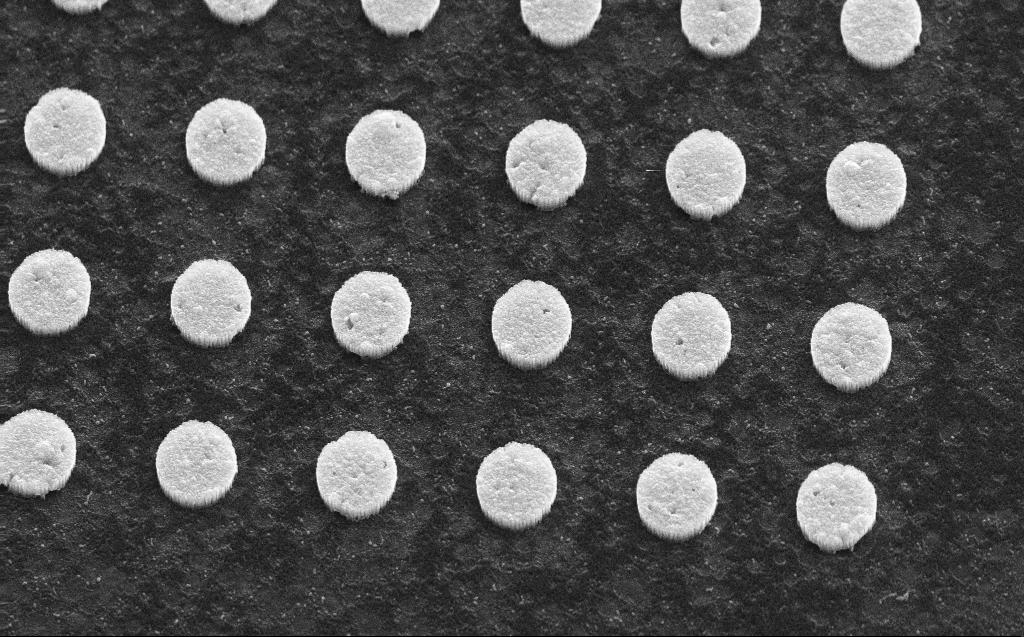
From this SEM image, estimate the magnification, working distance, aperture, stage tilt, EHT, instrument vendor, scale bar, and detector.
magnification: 30.14 K X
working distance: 5 mm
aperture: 30 µm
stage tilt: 45°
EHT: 30 kV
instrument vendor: Zeiss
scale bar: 1000 nm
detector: SE2